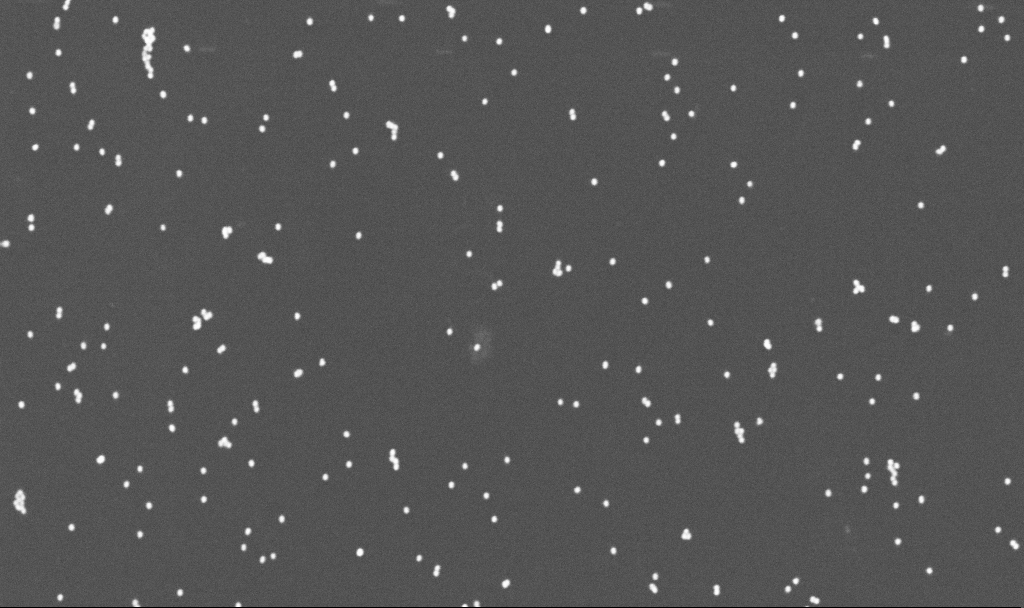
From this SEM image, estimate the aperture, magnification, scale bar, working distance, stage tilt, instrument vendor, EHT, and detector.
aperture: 30 µm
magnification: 70 K X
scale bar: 1000 nm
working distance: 3.3 mm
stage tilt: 0°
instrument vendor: Zeiss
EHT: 10 kV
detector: InLens